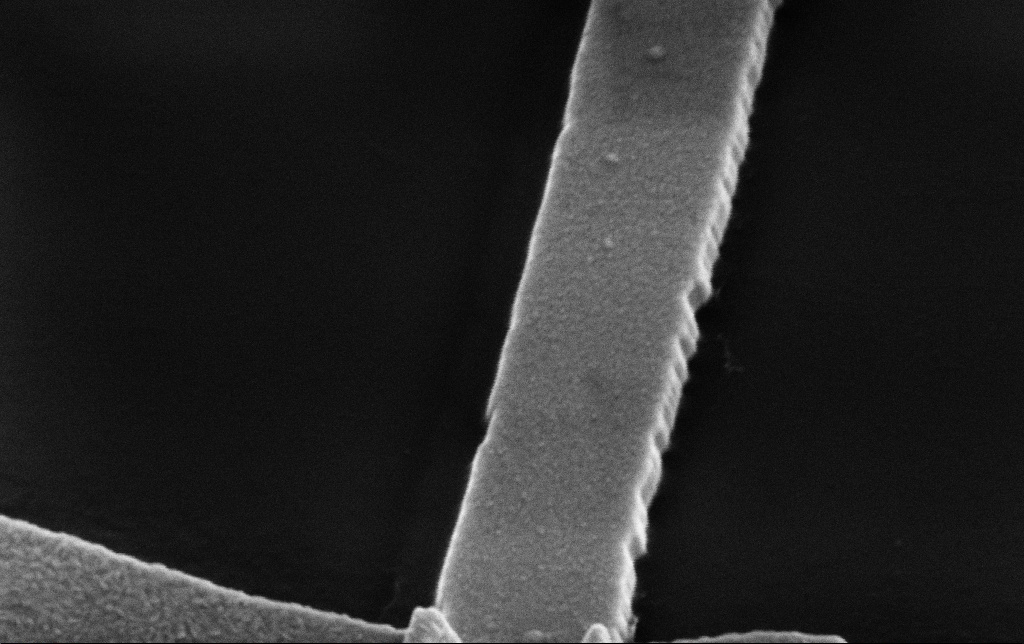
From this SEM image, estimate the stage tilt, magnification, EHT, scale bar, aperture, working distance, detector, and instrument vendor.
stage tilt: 45°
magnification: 100 K X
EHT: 5 kV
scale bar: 200 nm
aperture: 30 µm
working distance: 10.3 mm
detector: SE2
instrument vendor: Zeiss